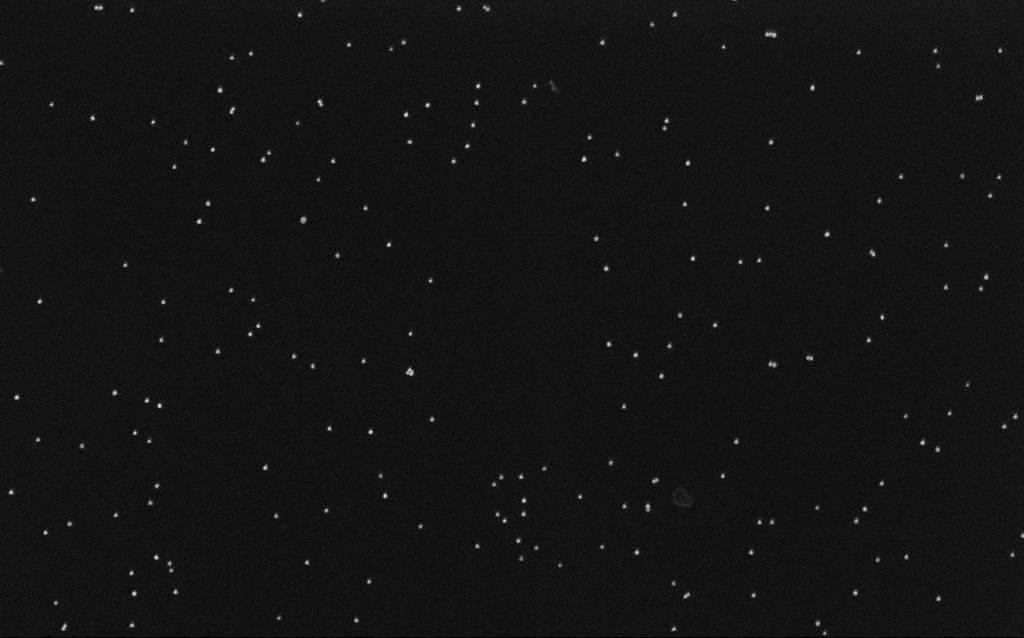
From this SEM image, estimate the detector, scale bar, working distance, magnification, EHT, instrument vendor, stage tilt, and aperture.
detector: InLens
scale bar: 200 nm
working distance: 6.5 mm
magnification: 100 K X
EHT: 10 kV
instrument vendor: Zeiss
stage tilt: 0°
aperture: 30 µm